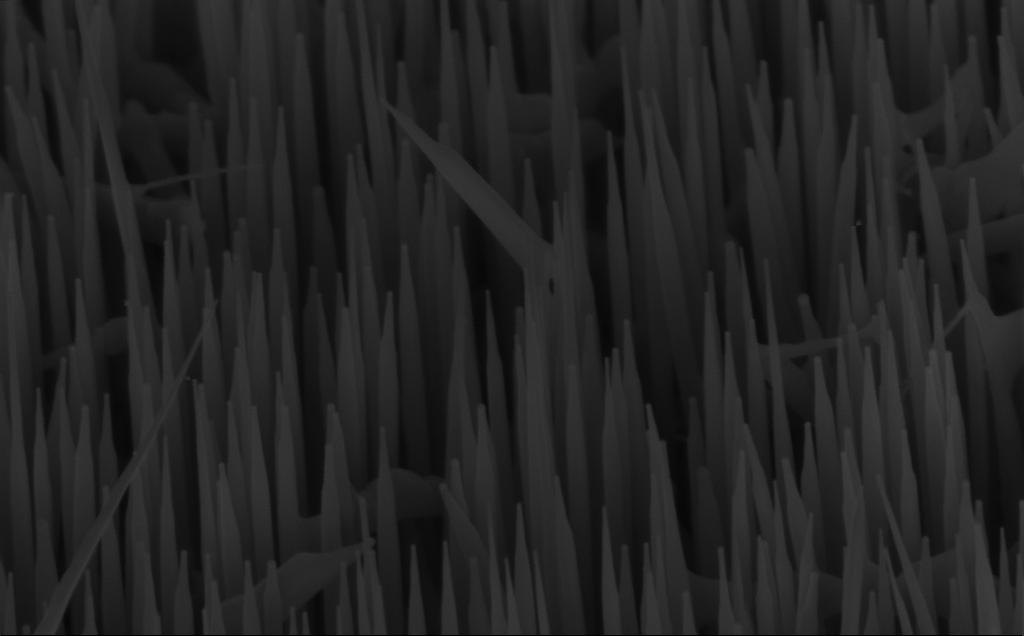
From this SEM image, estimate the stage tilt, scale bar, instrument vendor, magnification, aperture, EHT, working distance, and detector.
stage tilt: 45°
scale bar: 200 nm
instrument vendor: Zeiss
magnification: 80 K X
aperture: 30 µm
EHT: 5 kV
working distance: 6 mm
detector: InLens